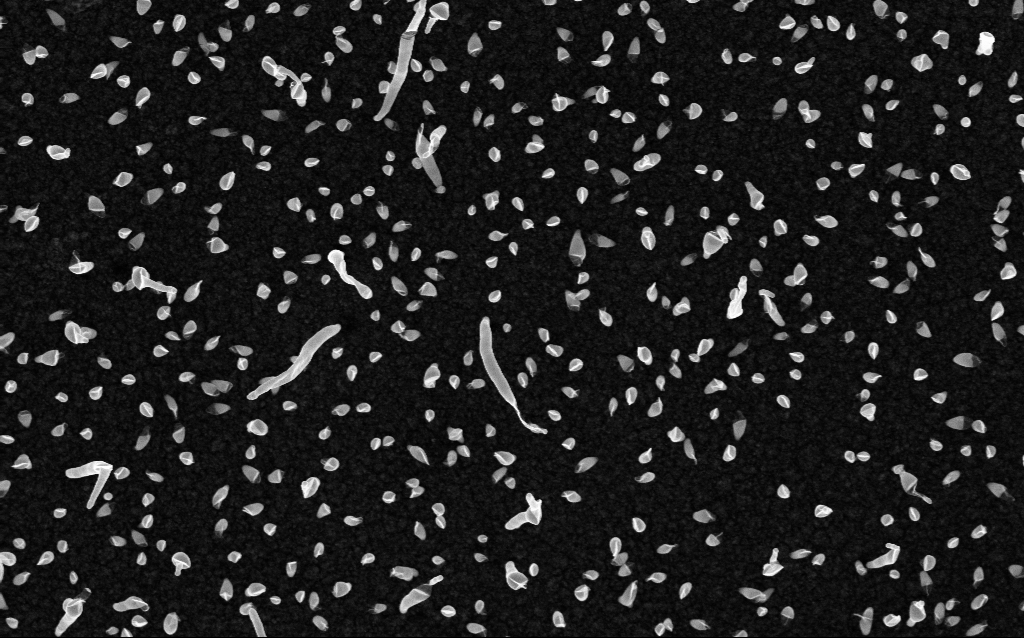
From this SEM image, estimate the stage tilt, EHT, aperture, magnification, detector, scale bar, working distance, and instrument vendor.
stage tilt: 0°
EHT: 5 kV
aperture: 30 µm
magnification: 50 K X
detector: InLens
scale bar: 1000 nm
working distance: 2.1 mm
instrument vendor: Zeiss